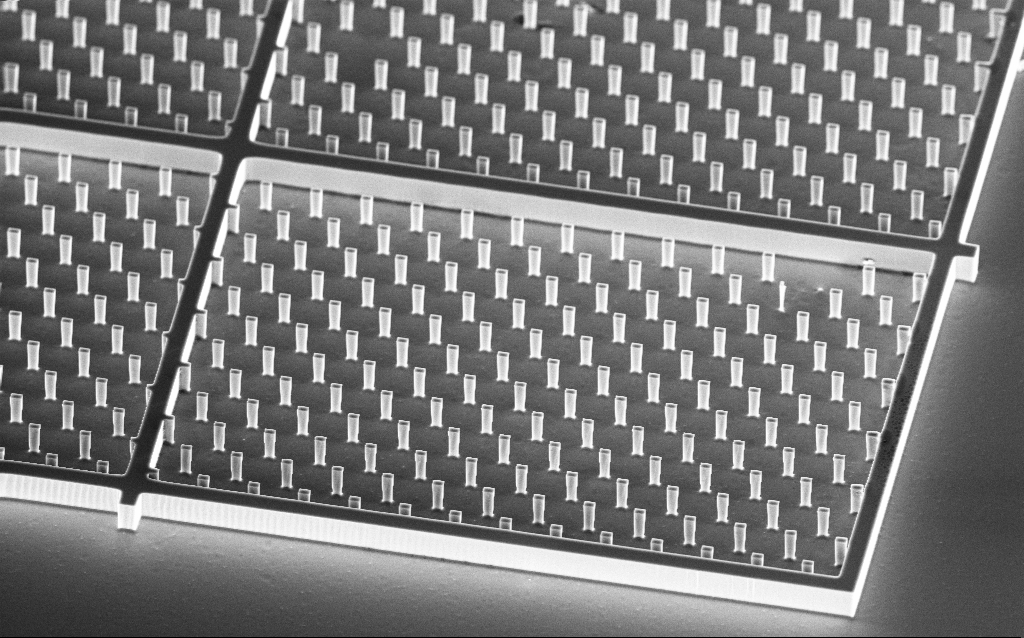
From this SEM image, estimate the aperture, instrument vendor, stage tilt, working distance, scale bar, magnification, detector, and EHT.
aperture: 30 µm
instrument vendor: Zeiss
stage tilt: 45°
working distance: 5.1 mm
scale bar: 10000 nm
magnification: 1.57 K X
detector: InLens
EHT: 10 kV